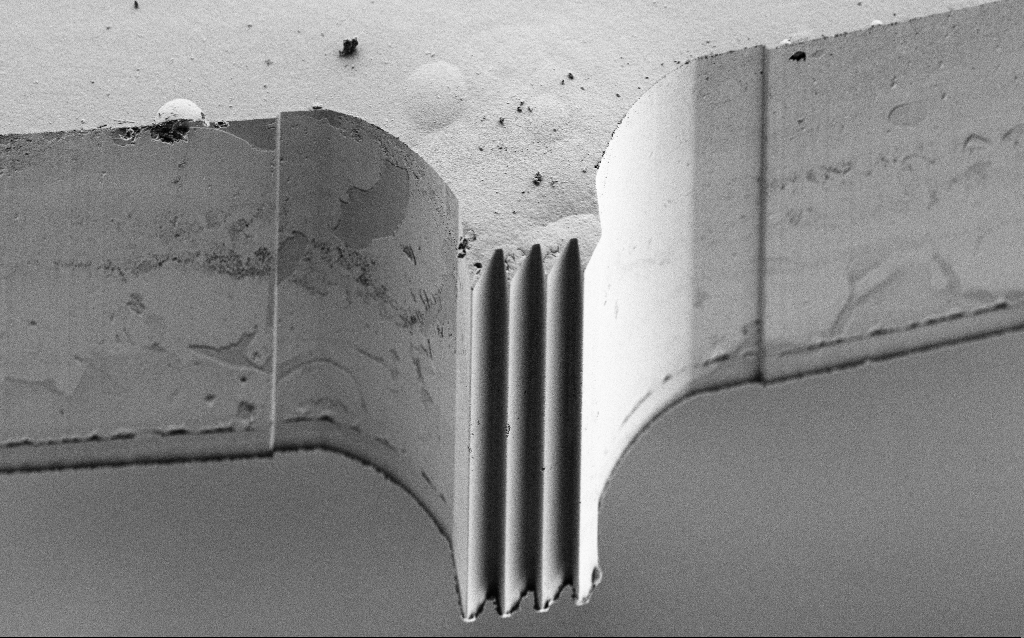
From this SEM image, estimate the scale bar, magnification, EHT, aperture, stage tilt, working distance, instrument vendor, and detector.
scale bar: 10000 nm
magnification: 1.23 K X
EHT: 3 kV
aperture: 30 µm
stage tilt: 45°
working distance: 4 mm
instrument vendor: Zeiss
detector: SE2